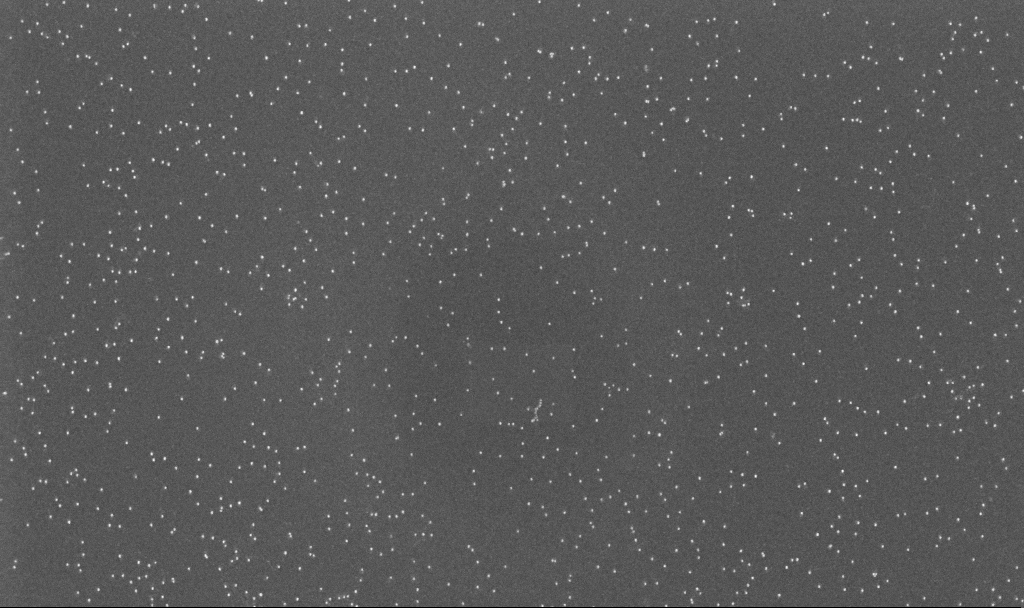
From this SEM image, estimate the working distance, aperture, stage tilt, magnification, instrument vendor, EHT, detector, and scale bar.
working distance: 3.2 mm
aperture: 30 µm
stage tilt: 0°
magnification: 70 K X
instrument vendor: Zeiss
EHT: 10 kV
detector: InLens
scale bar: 1000 nm